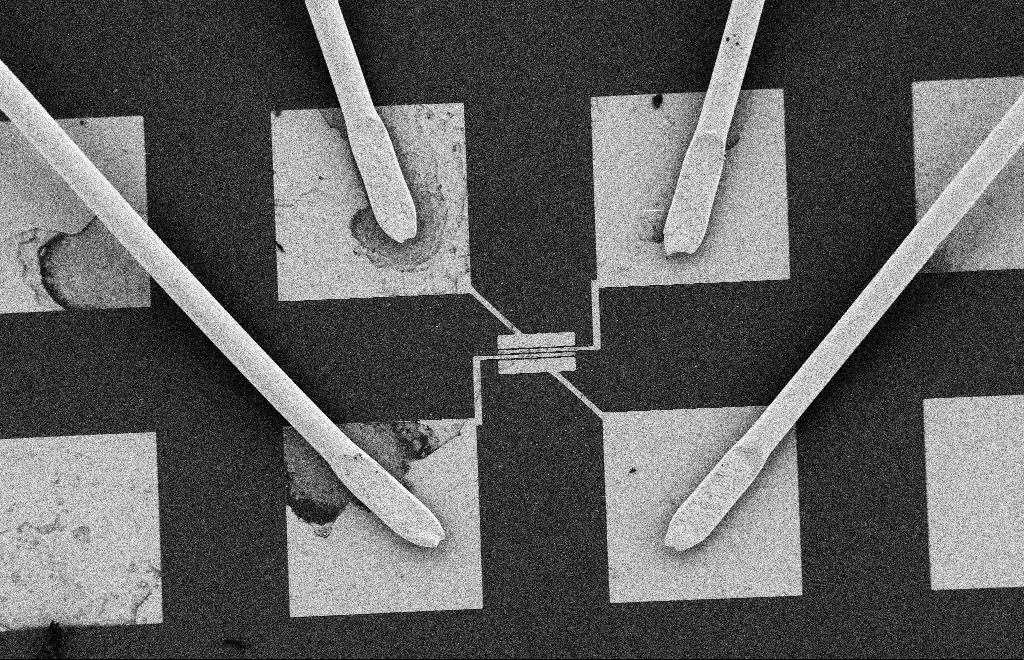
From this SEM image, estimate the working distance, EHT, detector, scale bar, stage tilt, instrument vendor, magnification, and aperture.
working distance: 9 mm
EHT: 2 kV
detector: SE2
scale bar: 100000 nm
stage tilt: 0°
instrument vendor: Zeiss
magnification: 0.463 K X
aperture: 20 µm